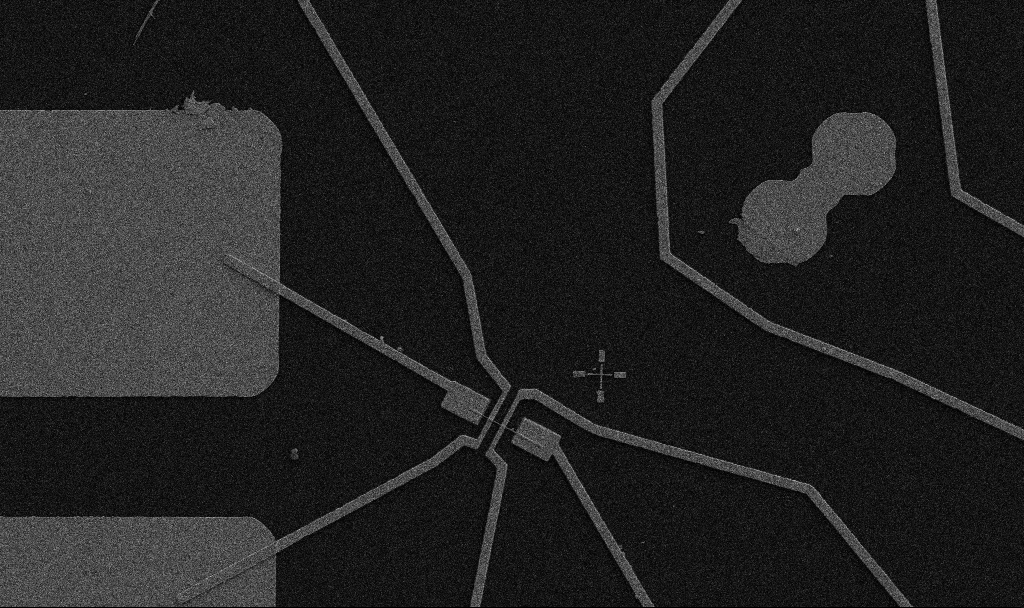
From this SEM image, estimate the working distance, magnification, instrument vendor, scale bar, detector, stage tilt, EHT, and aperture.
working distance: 10.7 mm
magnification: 5 K X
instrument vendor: Zeiss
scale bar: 10000 nm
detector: SE2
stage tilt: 0°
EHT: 5 kV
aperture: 30 µm